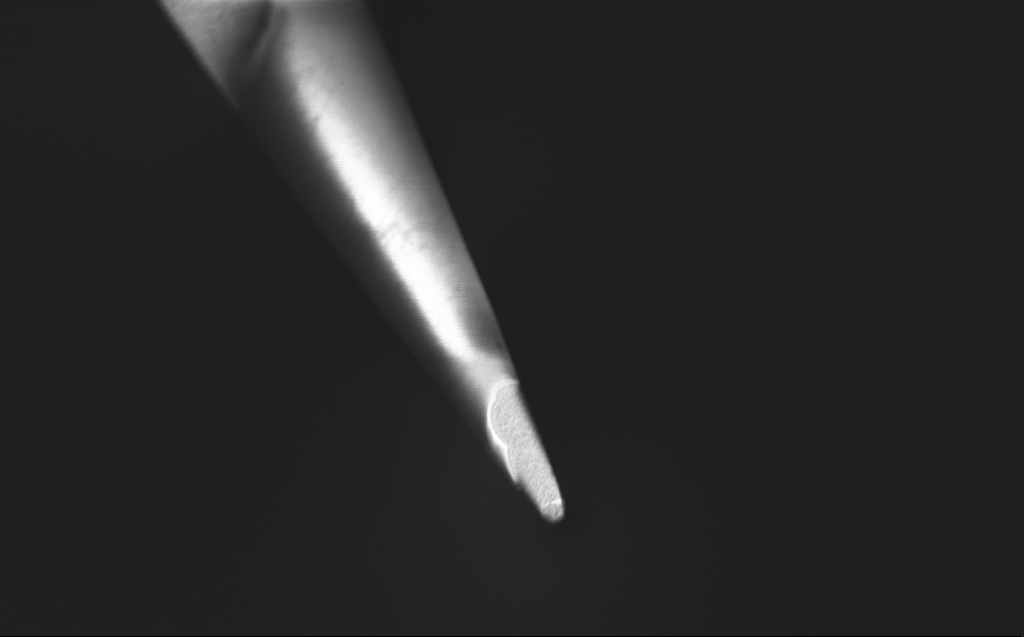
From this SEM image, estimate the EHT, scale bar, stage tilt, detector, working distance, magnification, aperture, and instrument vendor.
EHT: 0.8 kV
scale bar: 1000 nm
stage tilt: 45°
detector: InLens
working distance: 4 mm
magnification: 25 K X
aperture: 30 µm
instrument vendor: Zeiss